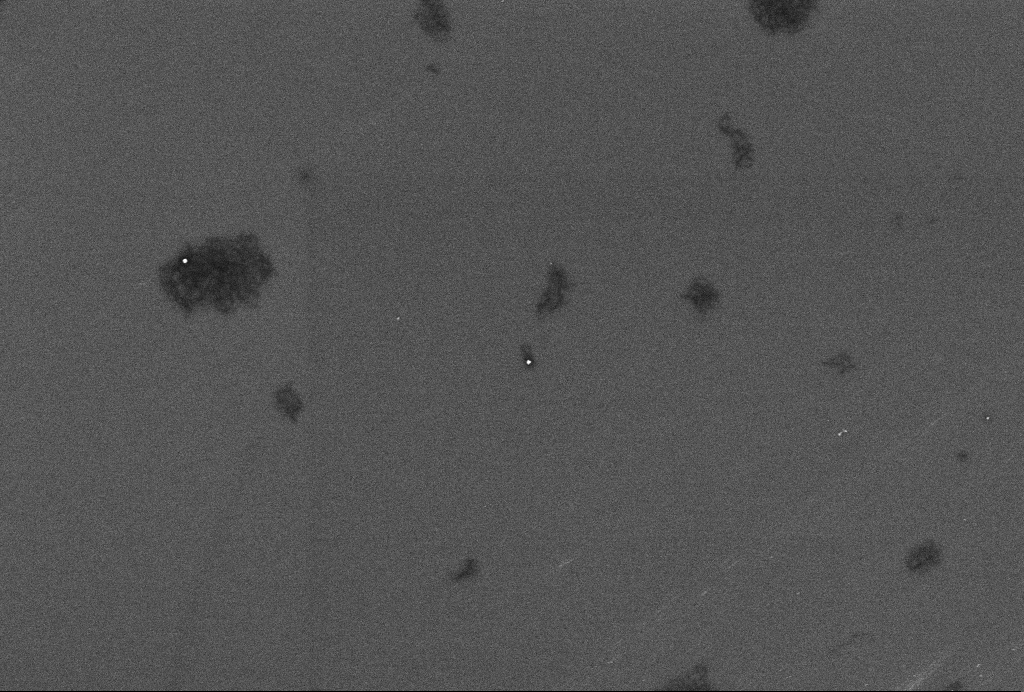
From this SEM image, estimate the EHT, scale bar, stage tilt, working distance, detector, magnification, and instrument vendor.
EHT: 2 kV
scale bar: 200 nm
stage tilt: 0°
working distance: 3.3 mm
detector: InLens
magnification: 60.8 K X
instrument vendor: Zeiss